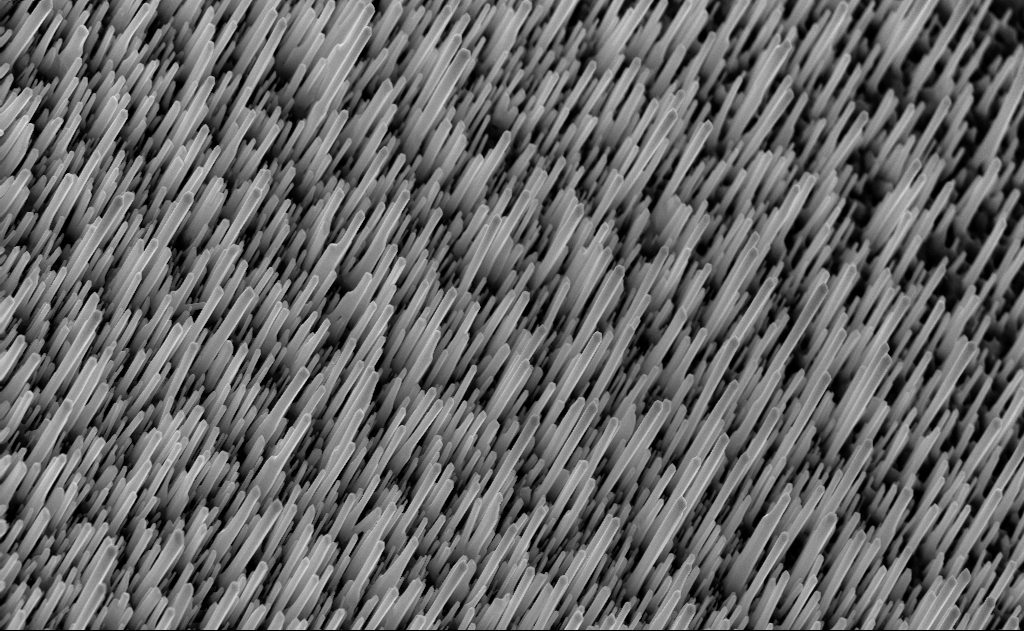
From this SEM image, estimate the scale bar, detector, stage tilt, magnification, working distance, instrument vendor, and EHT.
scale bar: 2000 nm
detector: InLens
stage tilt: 0°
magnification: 20 K X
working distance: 6 mm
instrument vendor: Zeiss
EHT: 10 kV